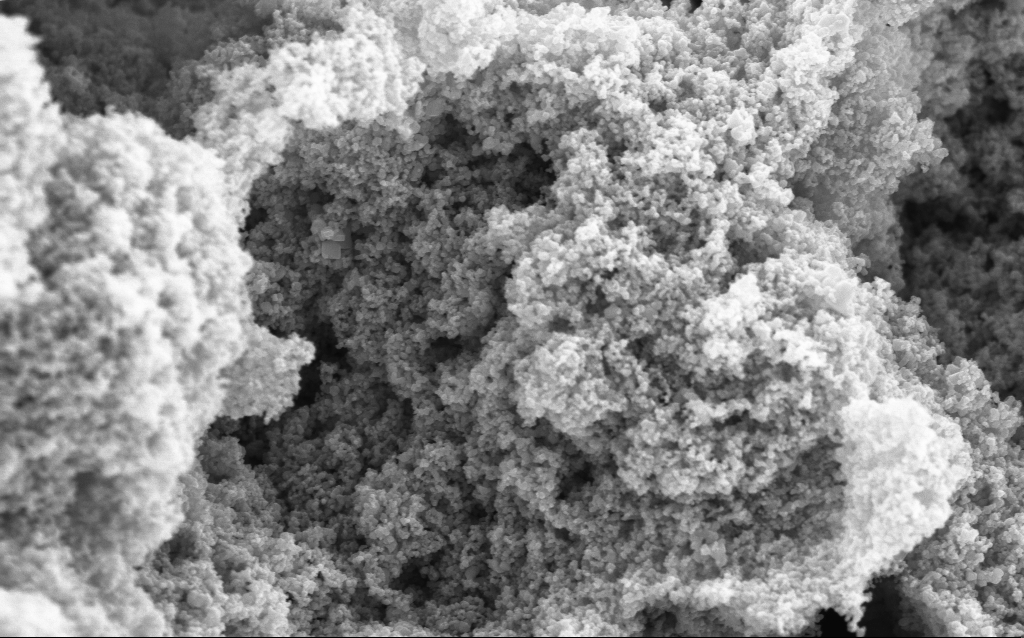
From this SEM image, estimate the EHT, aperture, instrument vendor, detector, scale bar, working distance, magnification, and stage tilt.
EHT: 5 kV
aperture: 30 µm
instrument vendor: Zeiss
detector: InLens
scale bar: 1000 nm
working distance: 4.4 mm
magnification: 68.66 K X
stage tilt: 0°